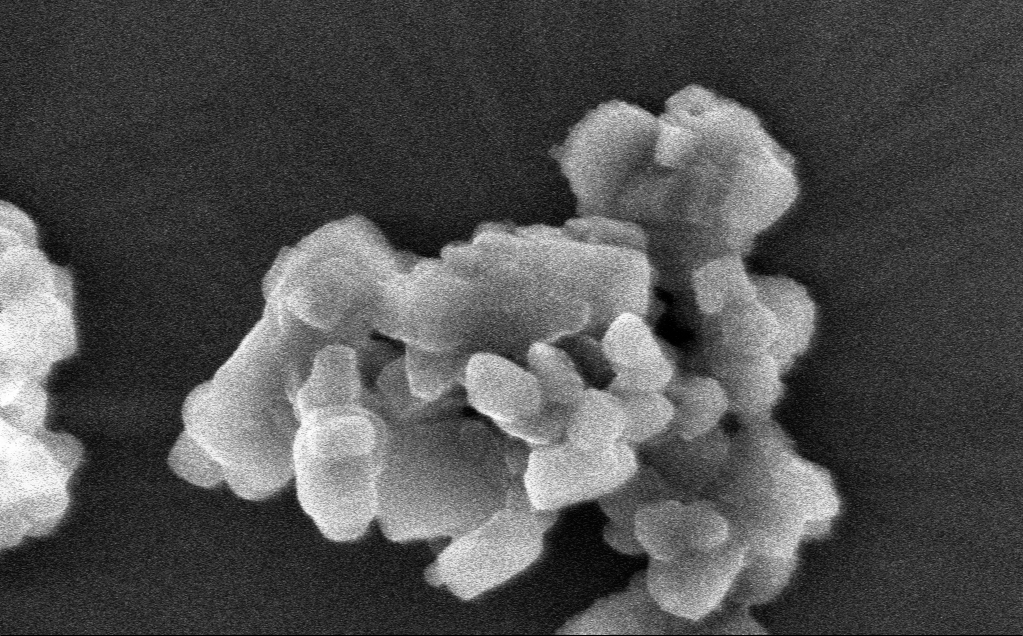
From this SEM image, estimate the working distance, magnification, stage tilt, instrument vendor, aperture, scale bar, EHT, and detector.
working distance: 3 mm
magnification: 225.14 K X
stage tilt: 0°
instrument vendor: Zeiss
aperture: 30 µm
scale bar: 200 nm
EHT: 3 kV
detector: InLens